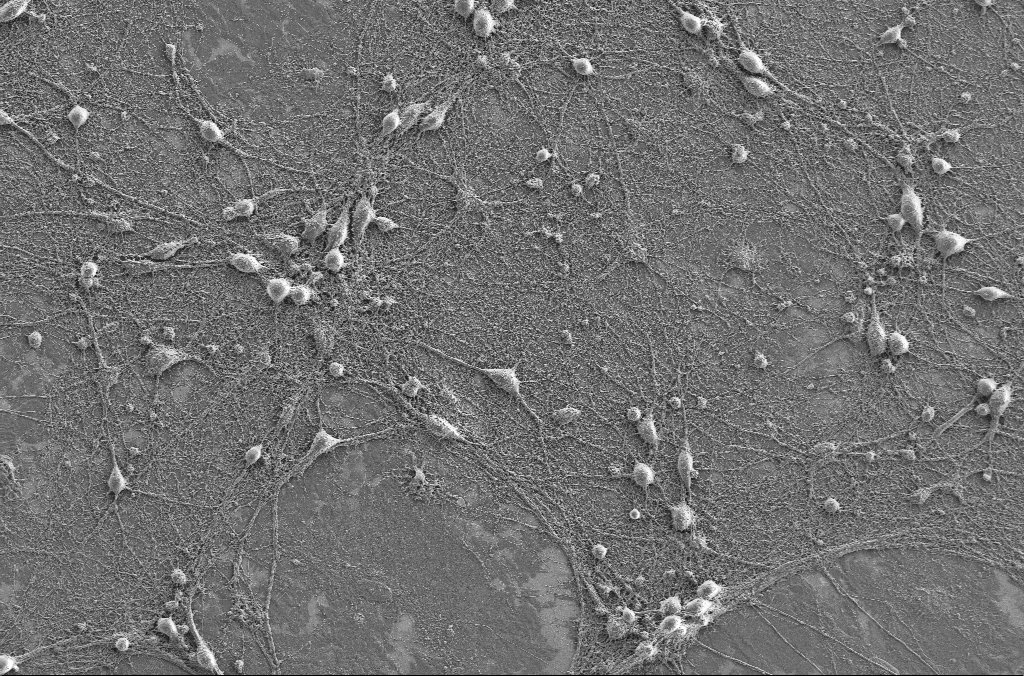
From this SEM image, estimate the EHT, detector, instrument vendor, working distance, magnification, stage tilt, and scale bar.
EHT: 2 kV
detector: SE2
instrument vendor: Zeiss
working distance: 4.1 mm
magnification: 1 K X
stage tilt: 0°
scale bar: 20000 nm